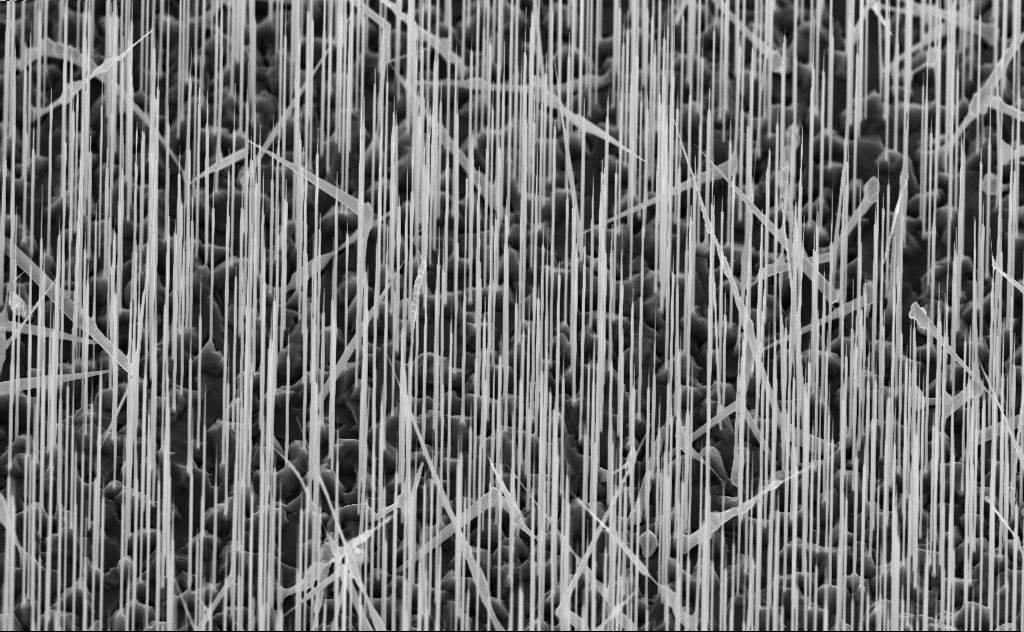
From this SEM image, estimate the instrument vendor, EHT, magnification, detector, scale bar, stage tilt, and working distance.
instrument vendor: Zeiss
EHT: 10 kV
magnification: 10 K X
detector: InLens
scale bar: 2000 nm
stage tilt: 45°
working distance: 6 mm